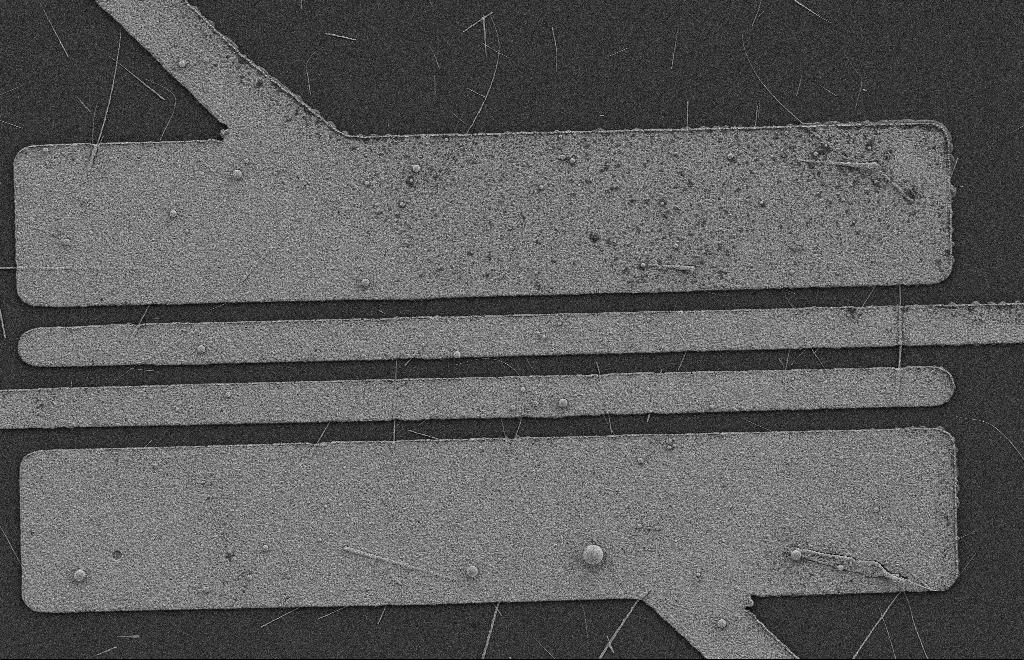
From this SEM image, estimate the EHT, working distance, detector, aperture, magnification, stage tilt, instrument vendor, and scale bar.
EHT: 2 kV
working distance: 9 mm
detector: SE2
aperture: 20 µm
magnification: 5.61 K X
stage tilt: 0°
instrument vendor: Zeiss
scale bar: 2000 nm